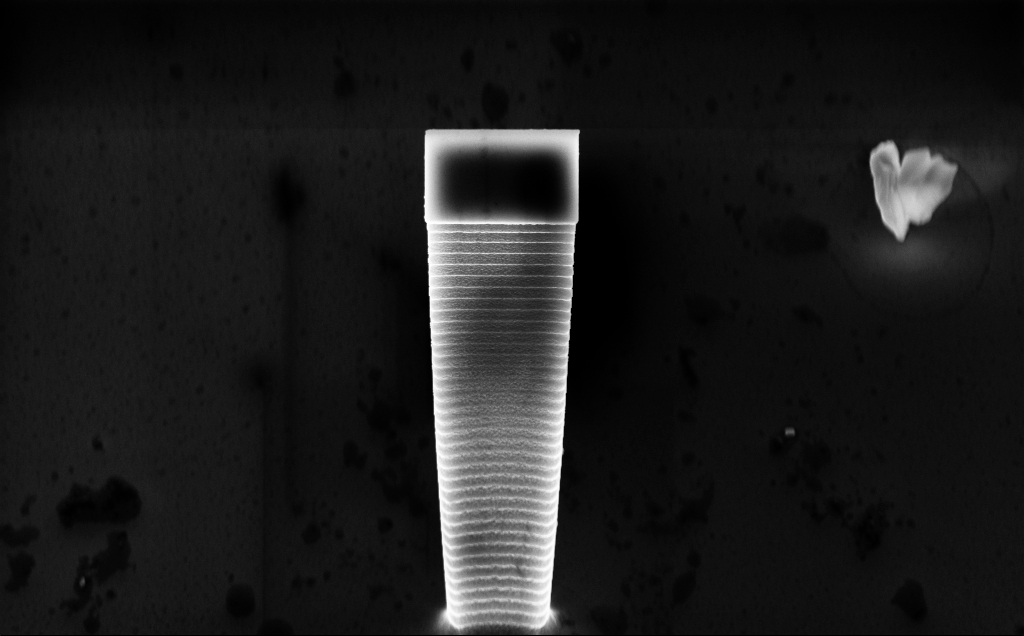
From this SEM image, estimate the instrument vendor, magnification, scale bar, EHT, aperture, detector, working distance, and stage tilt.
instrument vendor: Zeiss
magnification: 11.64 K X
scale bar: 2000 nm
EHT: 10 kV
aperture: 30 µm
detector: InLens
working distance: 5 mm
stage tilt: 45°